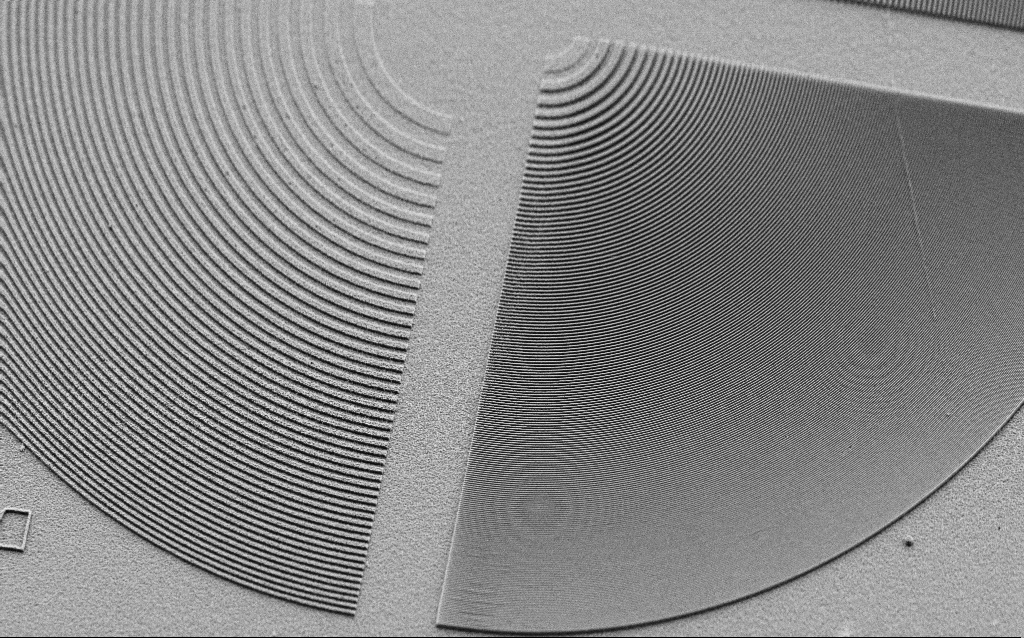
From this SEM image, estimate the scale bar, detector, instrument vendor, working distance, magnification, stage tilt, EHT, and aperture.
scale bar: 10000 nm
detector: SE2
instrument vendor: Zeiss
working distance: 5 mm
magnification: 3.02 K X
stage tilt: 45°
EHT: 3 kV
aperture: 30 µm